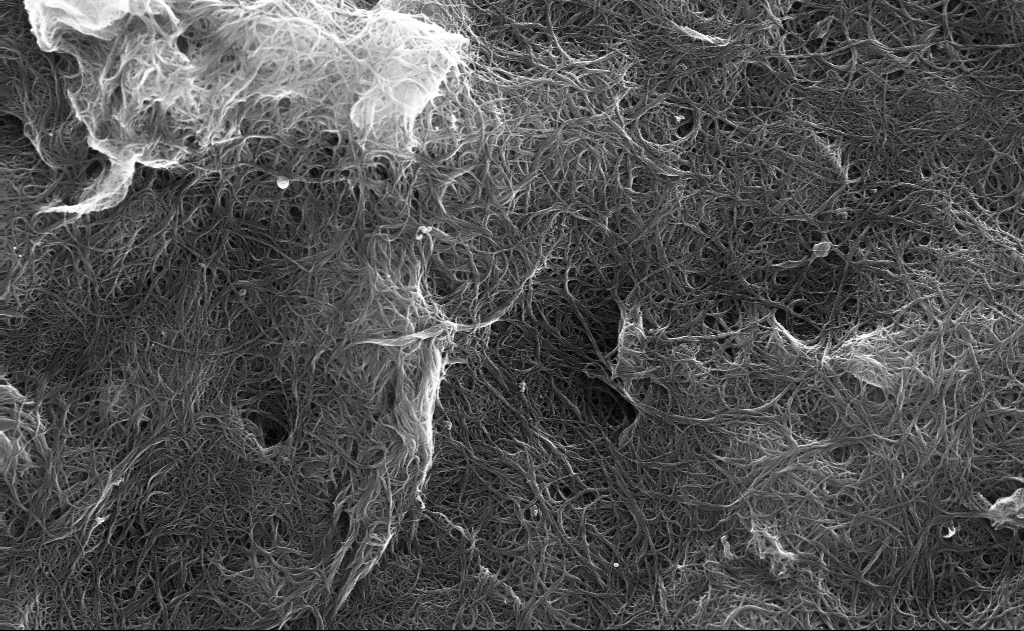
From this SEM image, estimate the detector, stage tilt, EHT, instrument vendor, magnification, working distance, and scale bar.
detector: InLens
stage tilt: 0°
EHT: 10 kV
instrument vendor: Zeiss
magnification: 27.47 K X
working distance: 3 mm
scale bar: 2000 nm